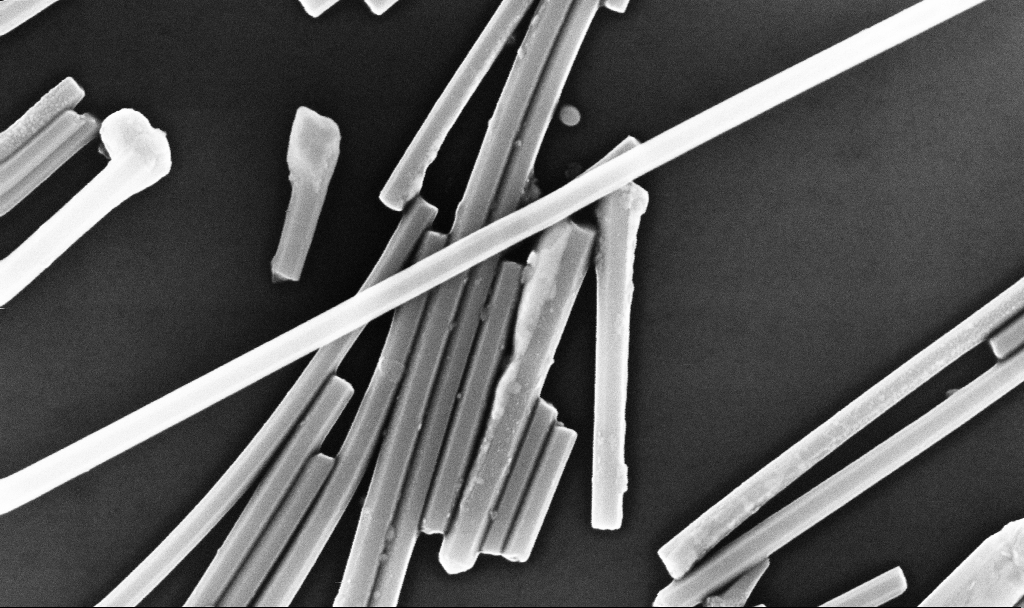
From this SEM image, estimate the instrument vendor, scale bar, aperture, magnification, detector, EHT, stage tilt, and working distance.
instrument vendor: Zeiss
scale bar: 200 nm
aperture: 30 µm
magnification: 100 K X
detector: InLens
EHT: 10 kV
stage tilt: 0°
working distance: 6.7 mm